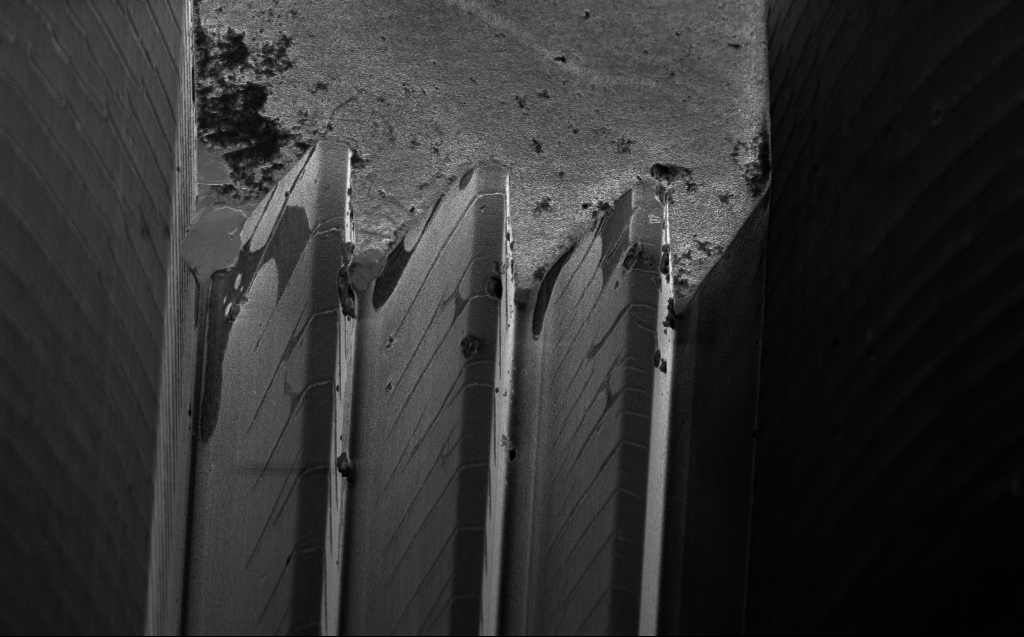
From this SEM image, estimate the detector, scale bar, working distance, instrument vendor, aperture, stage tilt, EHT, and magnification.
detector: InLens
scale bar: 10000 nm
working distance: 8 mm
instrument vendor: Zeiss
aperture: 30 µm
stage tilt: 45°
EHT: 3 kV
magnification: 5.15 K X